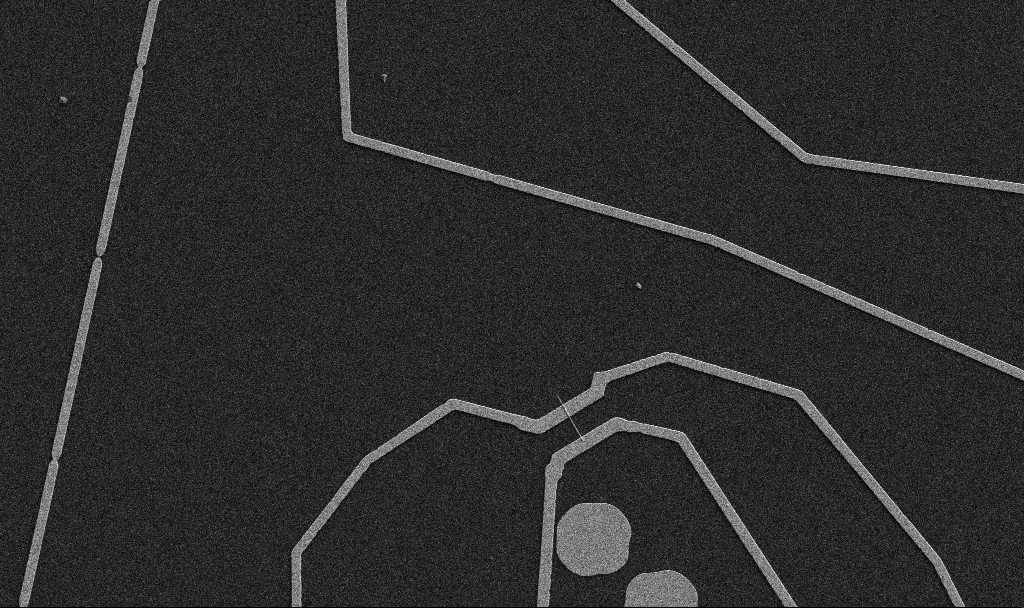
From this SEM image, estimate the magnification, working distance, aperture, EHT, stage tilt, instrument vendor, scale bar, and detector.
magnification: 5 K X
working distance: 10.7 mm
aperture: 30 µm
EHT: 5 kV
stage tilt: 0°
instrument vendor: Zeiss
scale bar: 10000 nm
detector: SE2